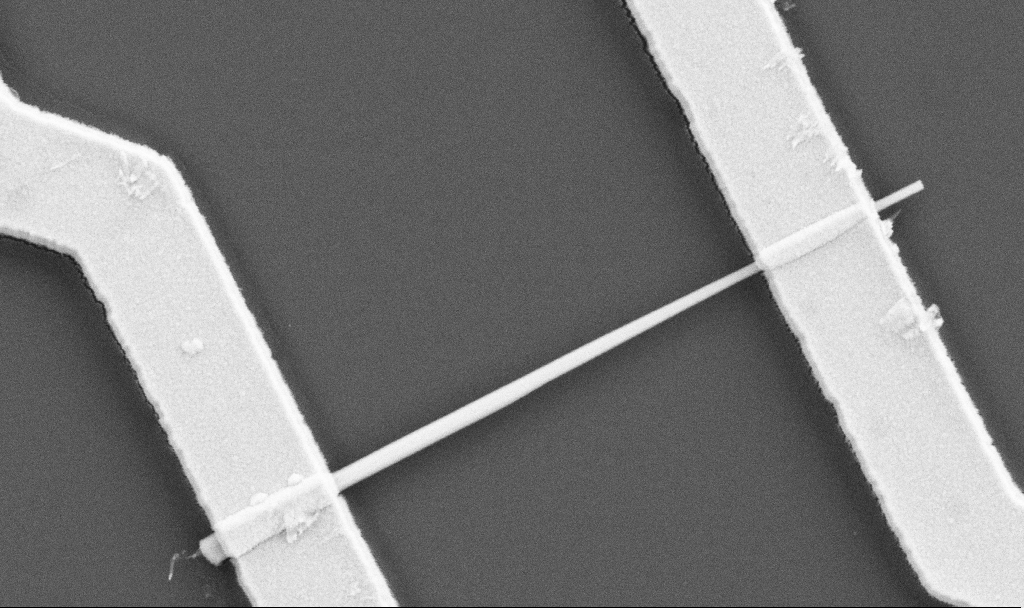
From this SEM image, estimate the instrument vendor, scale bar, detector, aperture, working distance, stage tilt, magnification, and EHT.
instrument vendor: Zeiss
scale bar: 1000 nm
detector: SE2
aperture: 30 µm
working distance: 10.7 mm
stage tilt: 0°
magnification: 49.93 K X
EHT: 5 kV